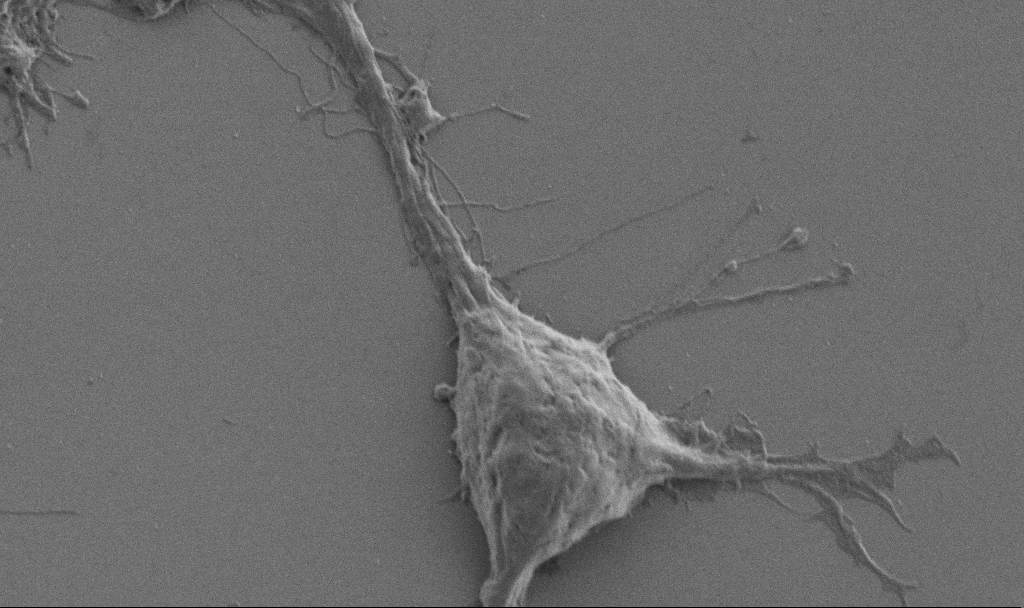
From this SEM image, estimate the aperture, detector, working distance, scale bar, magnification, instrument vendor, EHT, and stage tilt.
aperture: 30 µm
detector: SE2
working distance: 6.9 mm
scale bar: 2000 nm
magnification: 10 K X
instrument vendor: Zeiss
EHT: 1 kV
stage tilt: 0°